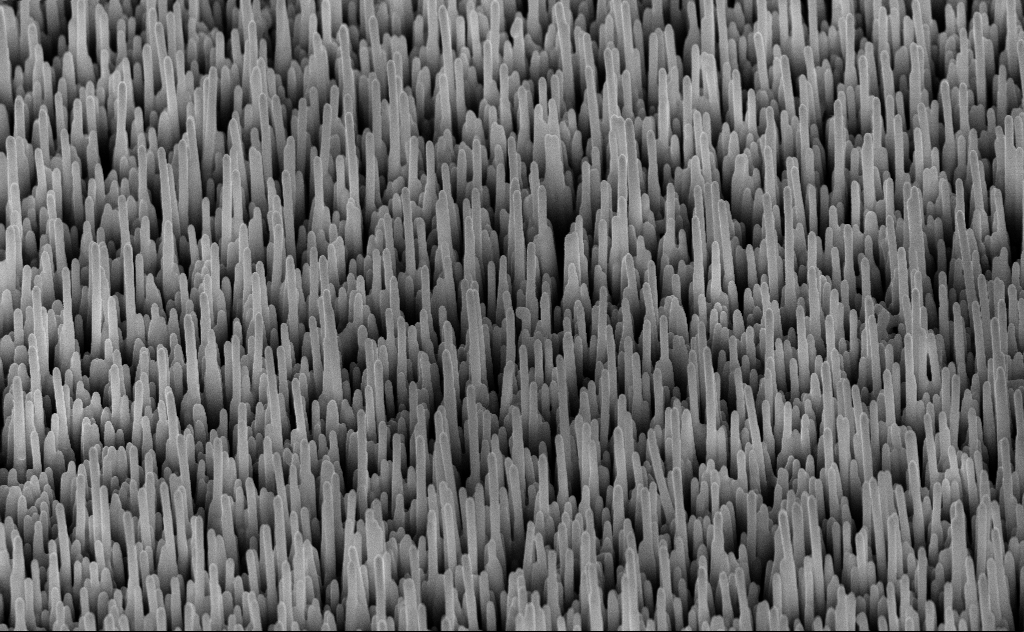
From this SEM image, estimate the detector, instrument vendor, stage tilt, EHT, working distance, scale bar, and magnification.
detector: InLens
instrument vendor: Zeiss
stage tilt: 45°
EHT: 10 kV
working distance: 8 mm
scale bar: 1000 nm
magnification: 20 K X